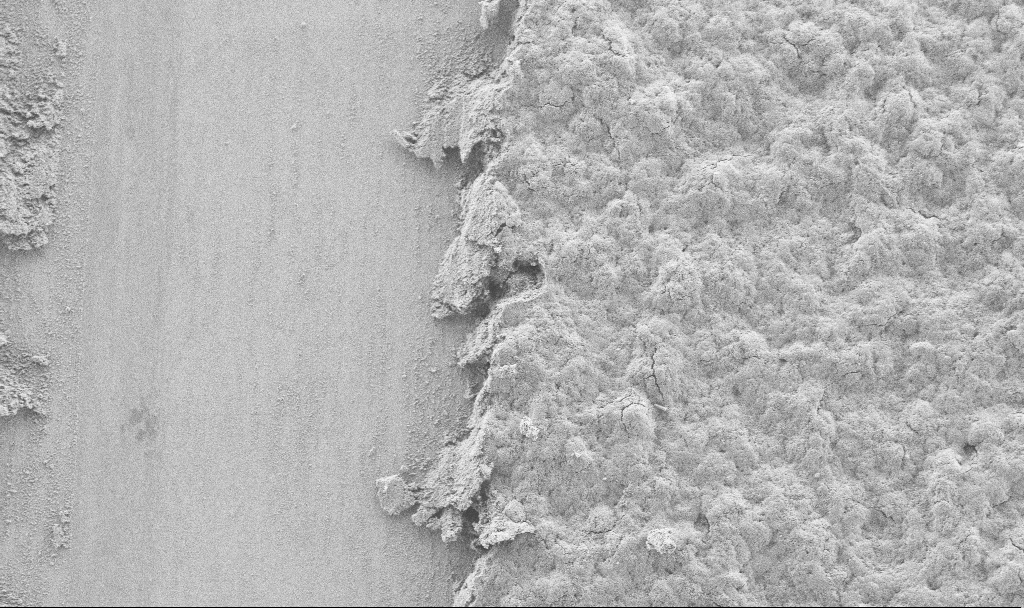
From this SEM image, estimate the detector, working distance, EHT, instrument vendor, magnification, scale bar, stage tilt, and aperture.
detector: SE2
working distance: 2.7 mm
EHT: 3 kV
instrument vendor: Zeiss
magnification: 1.09 K X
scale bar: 20000 nm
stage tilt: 0°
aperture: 30 µm